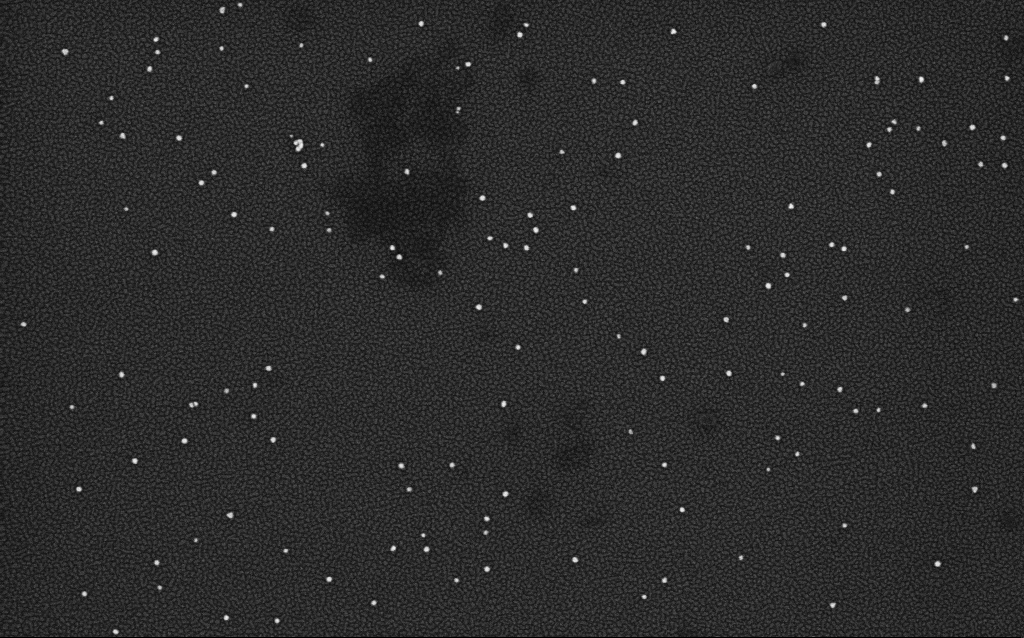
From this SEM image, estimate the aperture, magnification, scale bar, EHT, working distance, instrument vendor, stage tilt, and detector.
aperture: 30 µm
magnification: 100 K X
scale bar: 200 nm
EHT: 4 kV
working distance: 2.1 mm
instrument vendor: Zeiss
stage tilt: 0°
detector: InLens